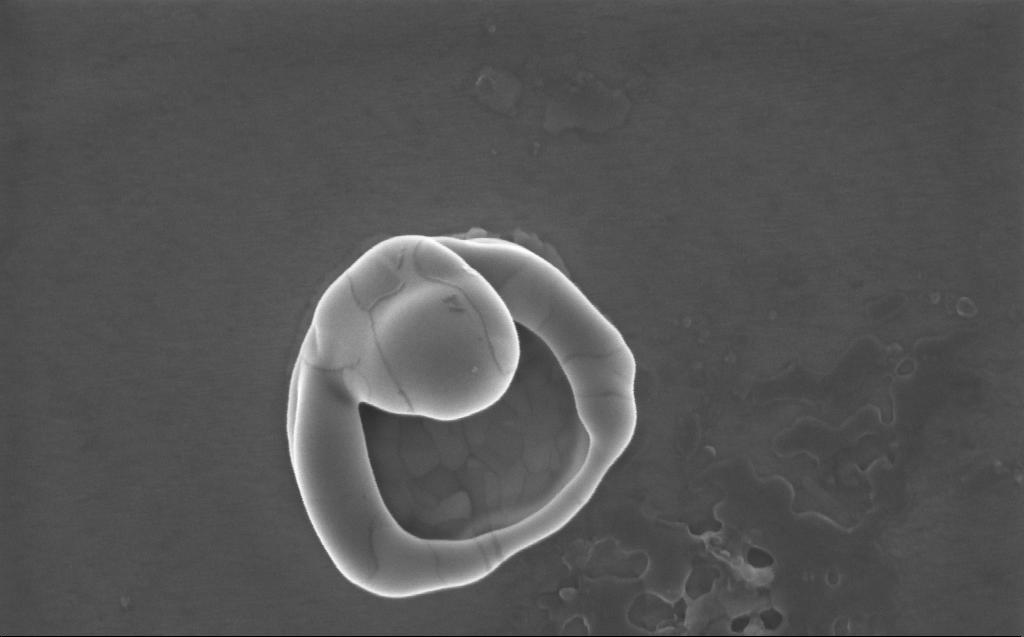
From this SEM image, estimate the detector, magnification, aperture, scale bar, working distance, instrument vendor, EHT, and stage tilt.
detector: InLens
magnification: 122 K X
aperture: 30 µm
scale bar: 200 nm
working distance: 2 mm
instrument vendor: Zeiss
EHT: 5 kV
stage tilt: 0°